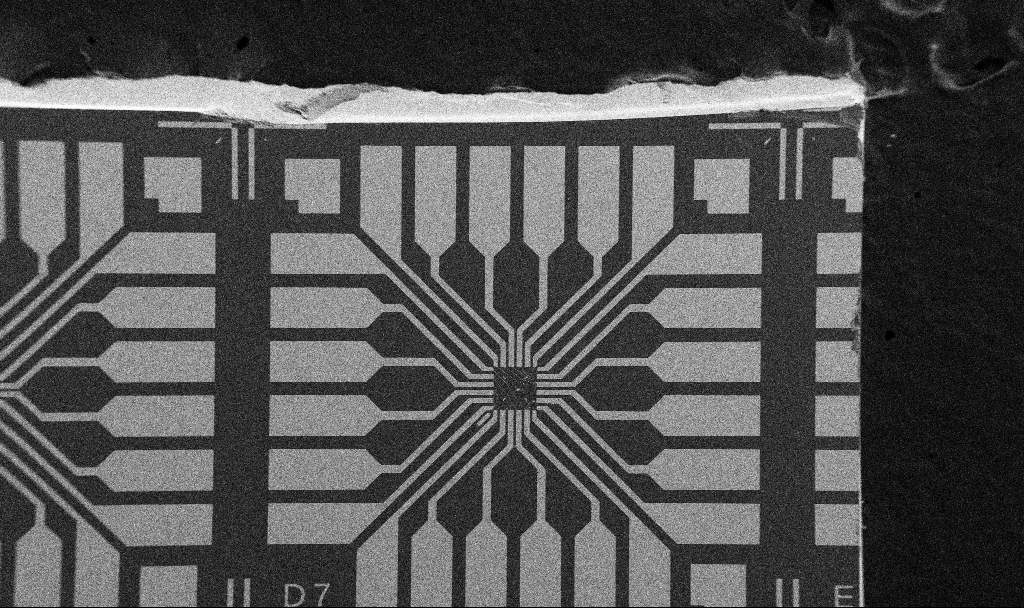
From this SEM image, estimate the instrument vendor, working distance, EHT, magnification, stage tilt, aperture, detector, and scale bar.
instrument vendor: Zeiss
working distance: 10.7 mm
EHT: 5 kV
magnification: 0.1 K X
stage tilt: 0°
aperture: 30 µm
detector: SE2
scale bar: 200000 nm